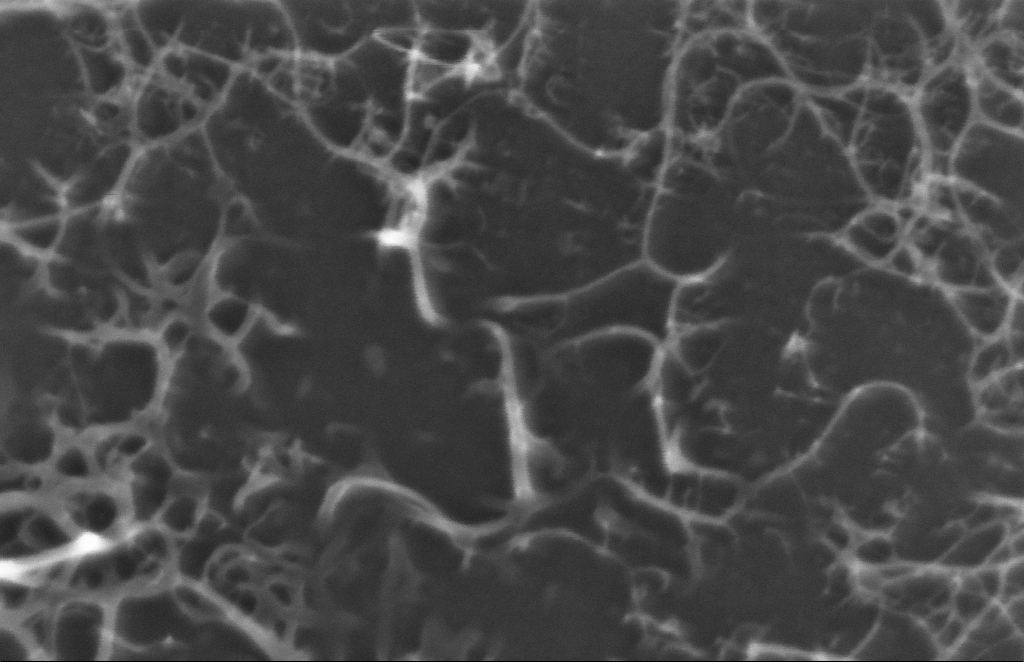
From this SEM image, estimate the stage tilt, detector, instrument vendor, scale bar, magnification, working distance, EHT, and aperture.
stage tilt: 0°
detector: InLens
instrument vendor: Zeiss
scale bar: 100 nm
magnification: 416.5 K X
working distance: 5 mm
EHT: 5 kV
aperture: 30 µm